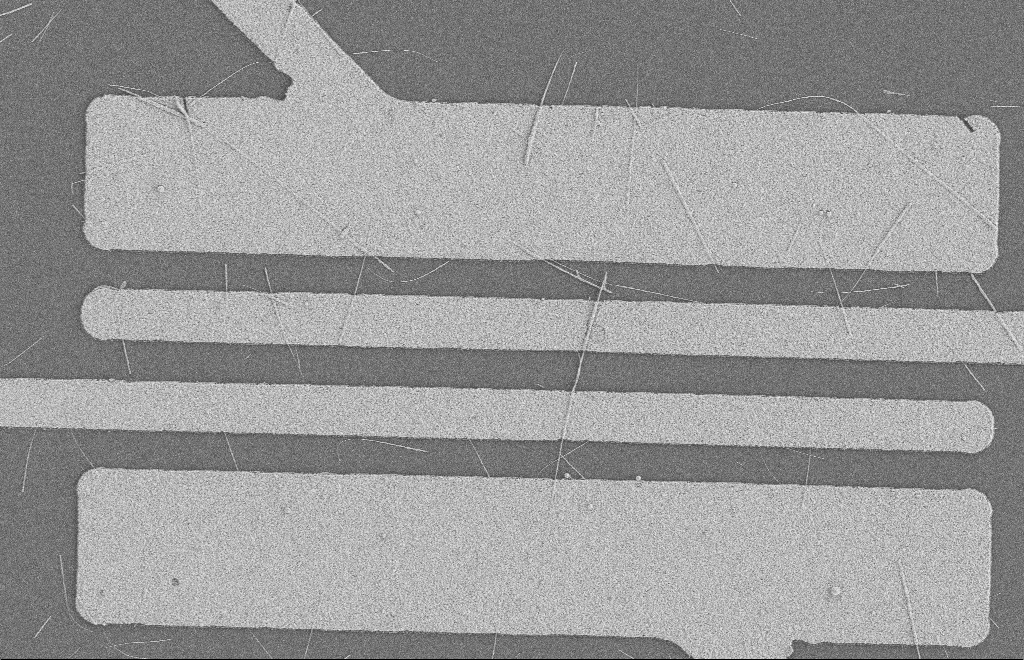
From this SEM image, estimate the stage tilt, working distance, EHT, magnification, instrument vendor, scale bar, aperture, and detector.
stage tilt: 0°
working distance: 8 mm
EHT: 2 kV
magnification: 5.5 K X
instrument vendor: Zeiss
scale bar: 2000 nm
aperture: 20 µm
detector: SE2